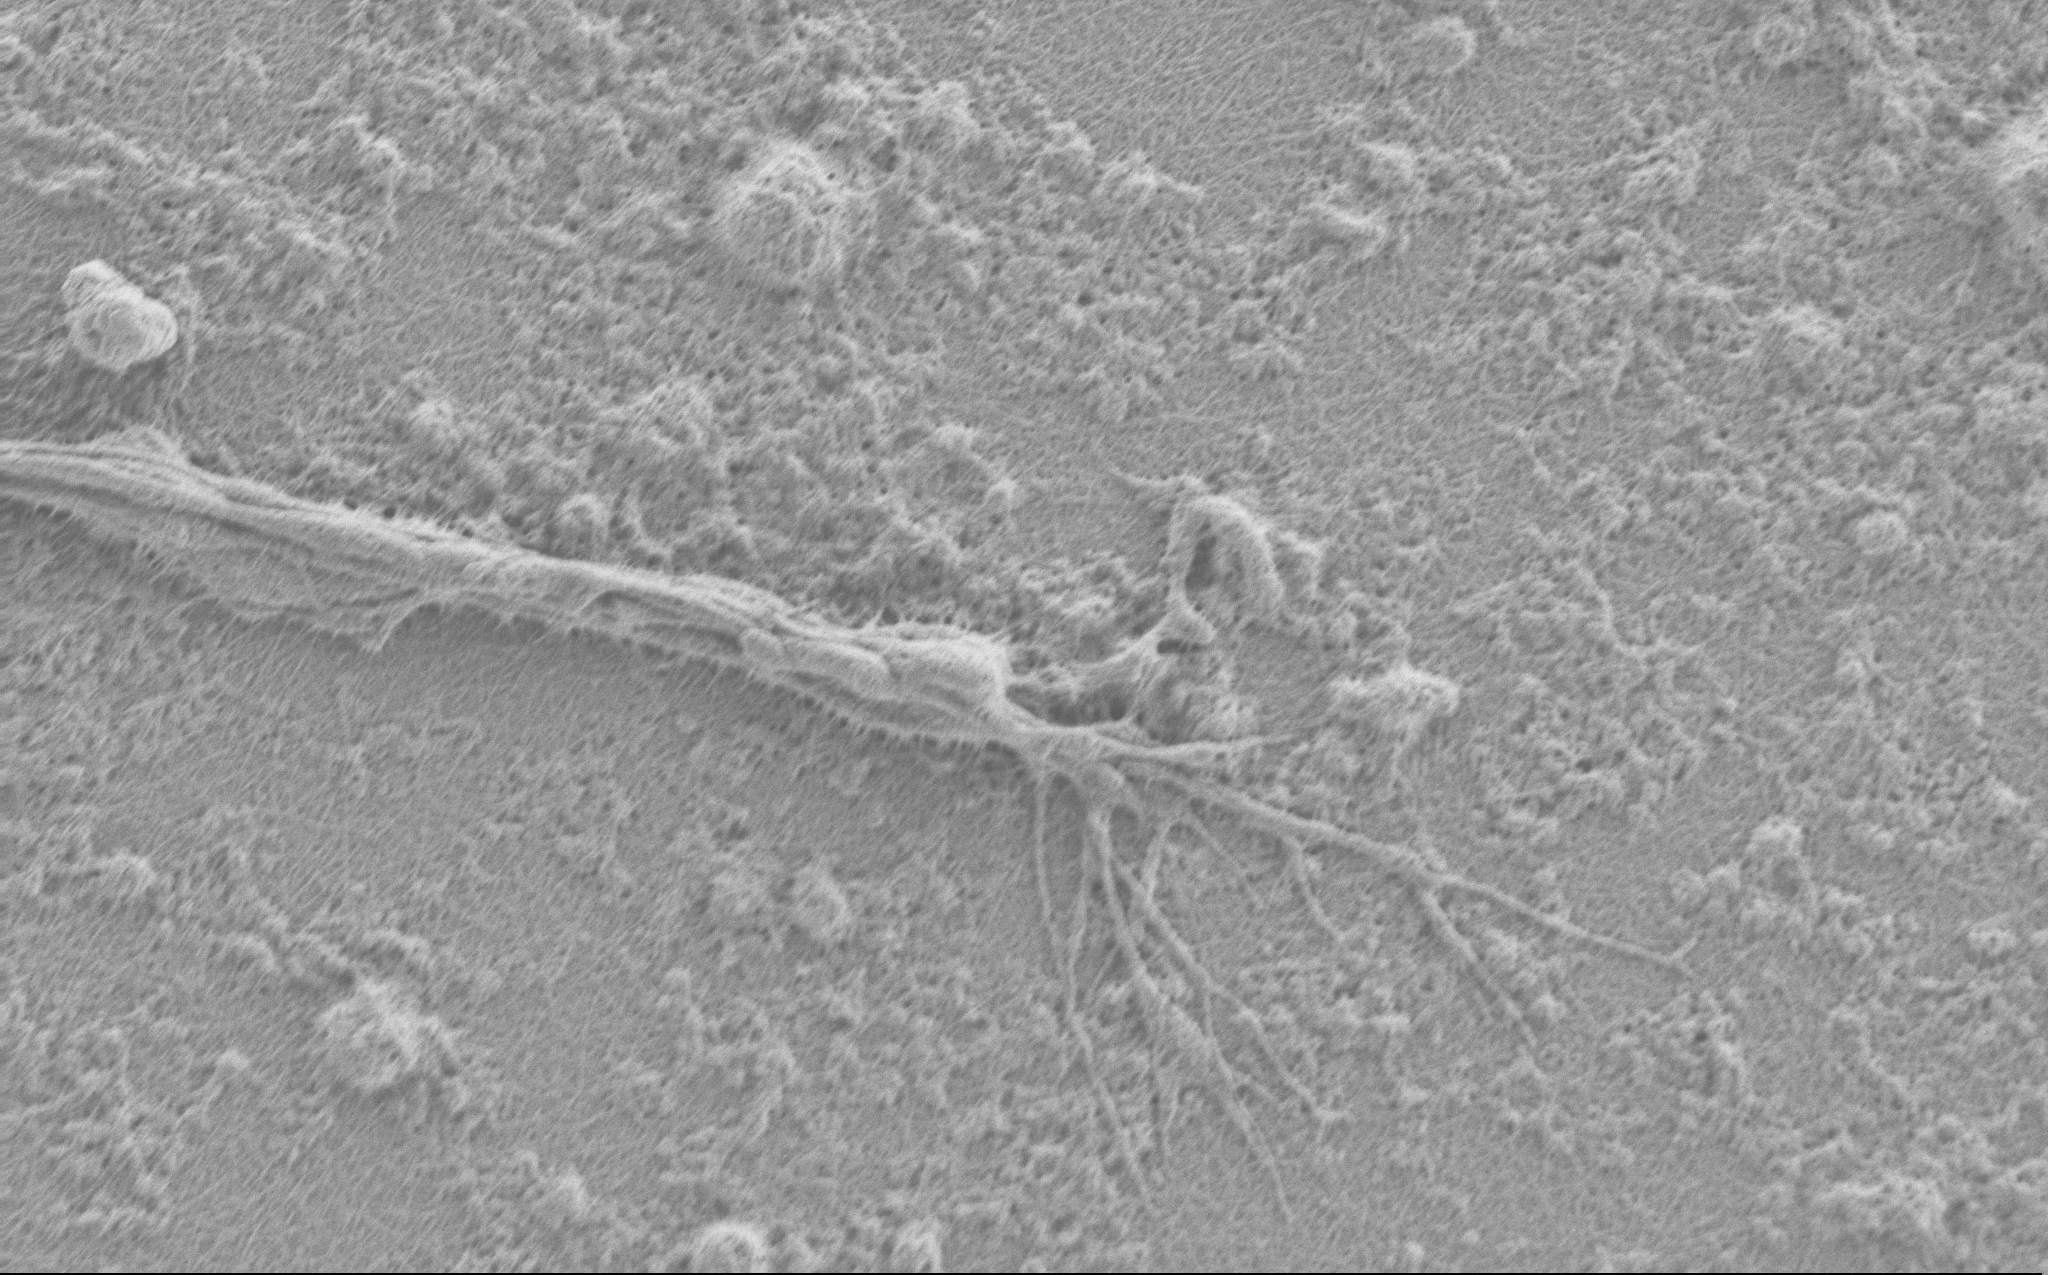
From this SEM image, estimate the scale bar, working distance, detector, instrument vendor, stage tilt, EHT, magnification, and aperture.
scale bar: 2000 nm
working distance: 4 mm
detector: SE2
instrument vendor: Zeiss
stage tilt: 0°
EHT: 0.9 kV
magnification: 10 K X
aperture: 30 µm